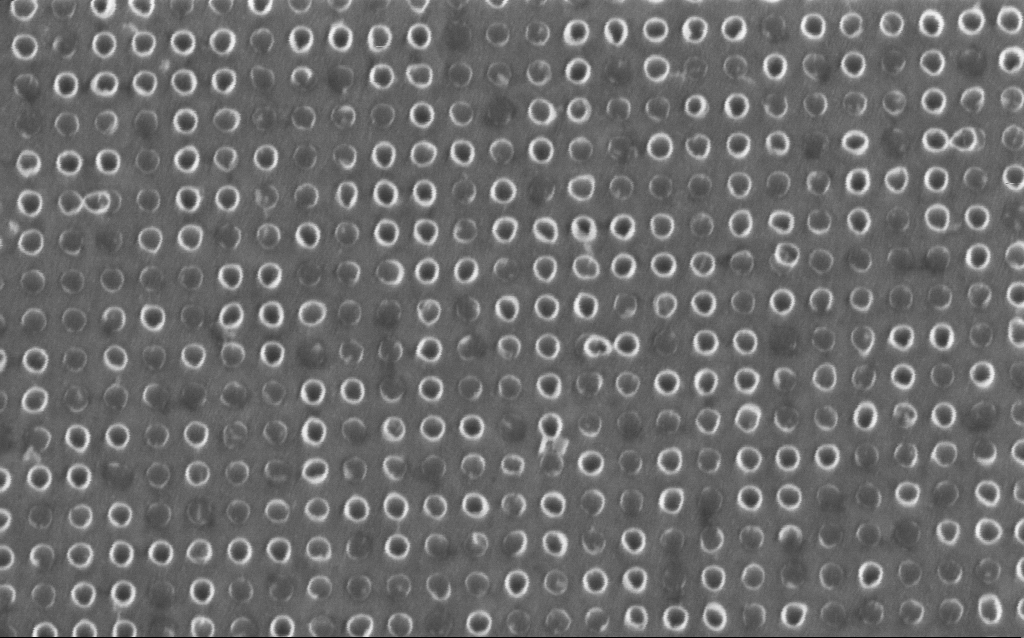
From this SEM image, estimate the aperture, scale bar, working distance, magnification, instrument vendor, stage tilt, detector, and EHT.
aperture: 30 µm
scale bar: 200 nm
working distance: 5.9 mm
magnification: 102.48 K X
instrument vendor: Zeiss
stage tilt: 0°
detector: InLens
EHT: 1.5 kV